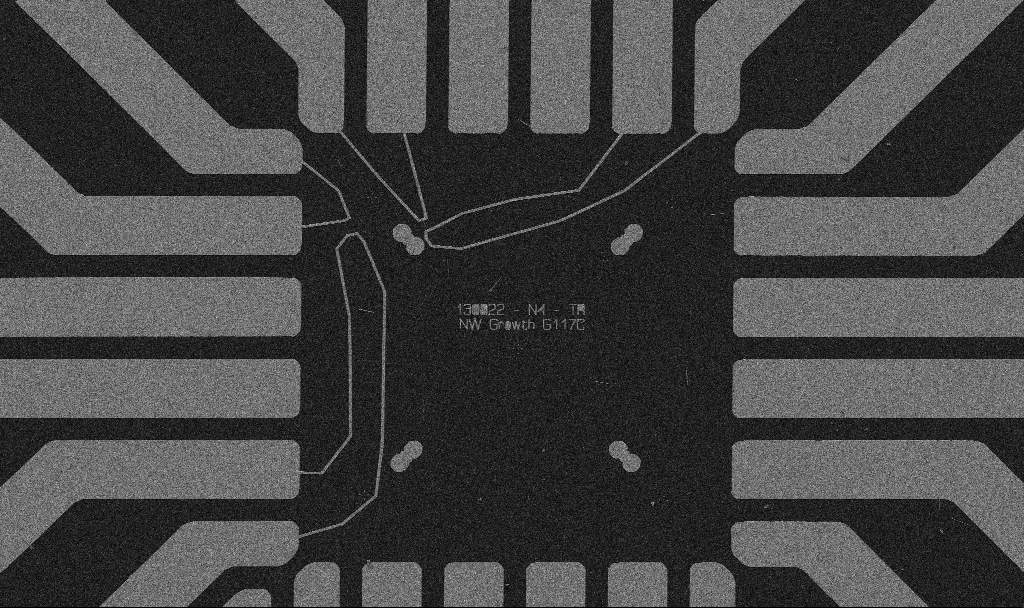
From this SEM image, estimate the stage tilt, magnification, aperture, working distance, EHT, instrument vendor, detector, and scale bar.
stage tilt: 0°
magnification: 1 K X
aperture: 30 µm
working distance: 10.7 mm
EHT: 5 kV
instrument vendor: Zeiss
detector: SE2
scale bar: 20000 nm